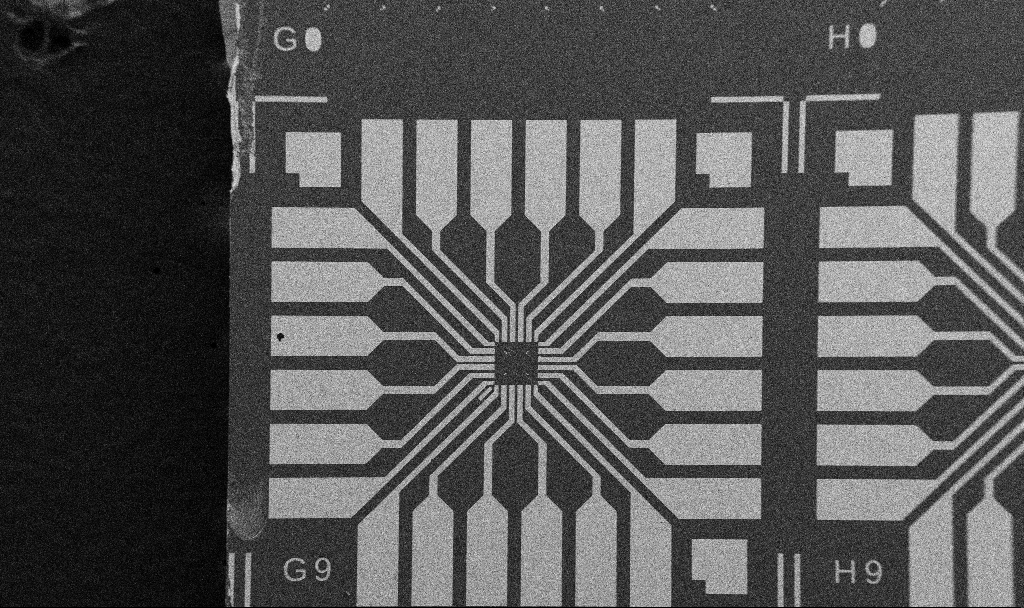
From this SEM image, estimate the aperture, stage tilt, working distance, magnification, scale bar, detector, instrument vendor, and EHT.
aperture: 30 µm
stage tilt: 0°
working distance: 10.7 mm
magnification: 0.1 K X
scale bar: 200000 nm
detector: SE2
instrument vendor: Zeiss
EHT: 5 kV